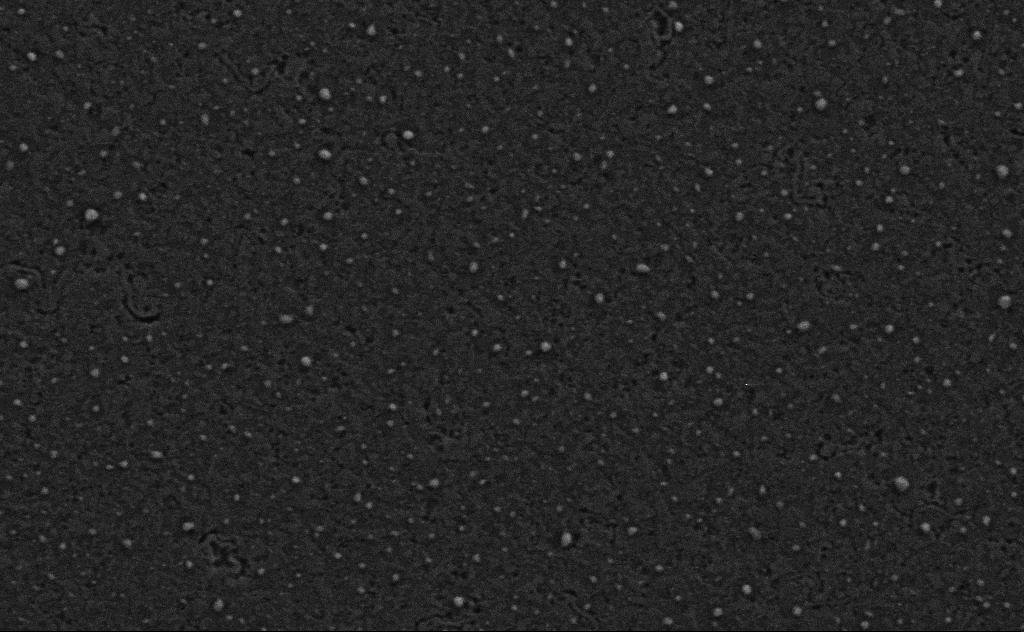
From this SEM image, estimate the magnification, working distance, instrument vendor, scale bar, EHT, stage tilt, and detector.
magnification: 80 K X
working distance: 4 mm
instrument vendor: Zeiss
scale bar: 200 nm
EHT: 3 kV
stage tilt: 0°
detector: SE2